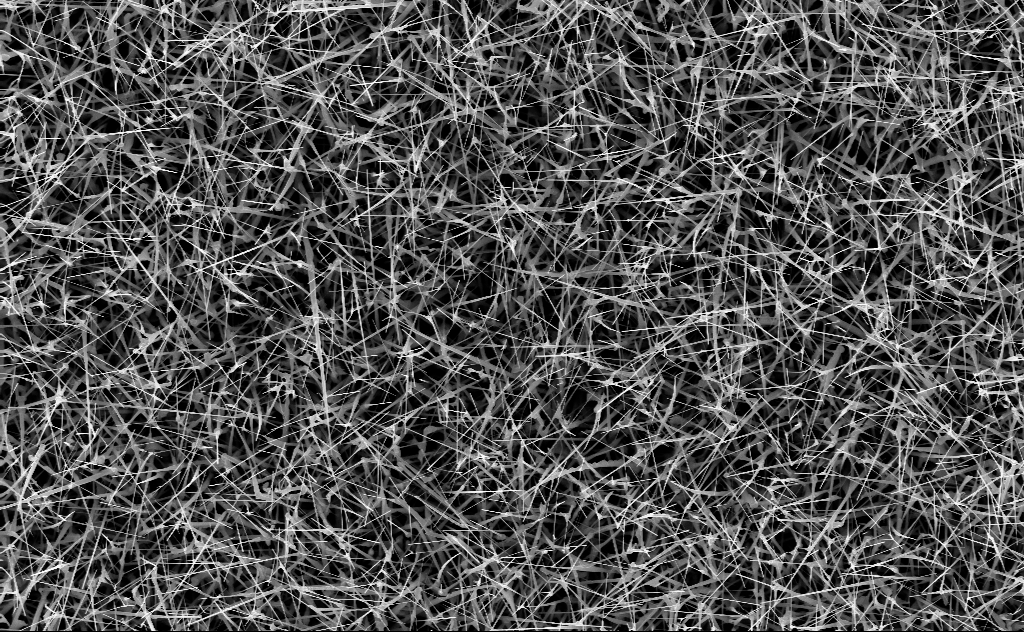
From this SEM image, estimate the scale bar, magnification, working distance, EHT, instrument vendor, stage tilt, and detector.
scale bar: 2000 nm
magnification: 10 K X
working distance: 6 mm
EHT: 10 kV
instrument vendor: Zeiss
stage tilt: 0°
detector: InLens